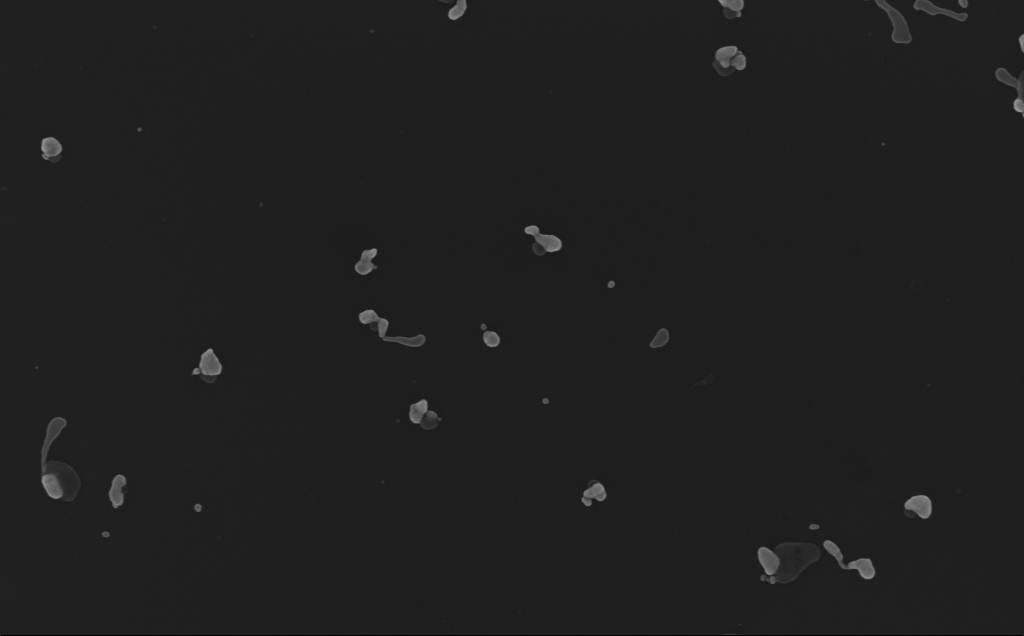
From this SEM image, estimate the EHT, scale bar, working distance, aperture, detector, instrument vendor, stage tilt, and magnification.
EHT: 10 kV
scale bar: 200 nm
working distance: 3 mm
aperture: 30 µm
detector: InLens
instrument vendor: Zeiss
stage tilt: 0°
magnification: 106.94 K X